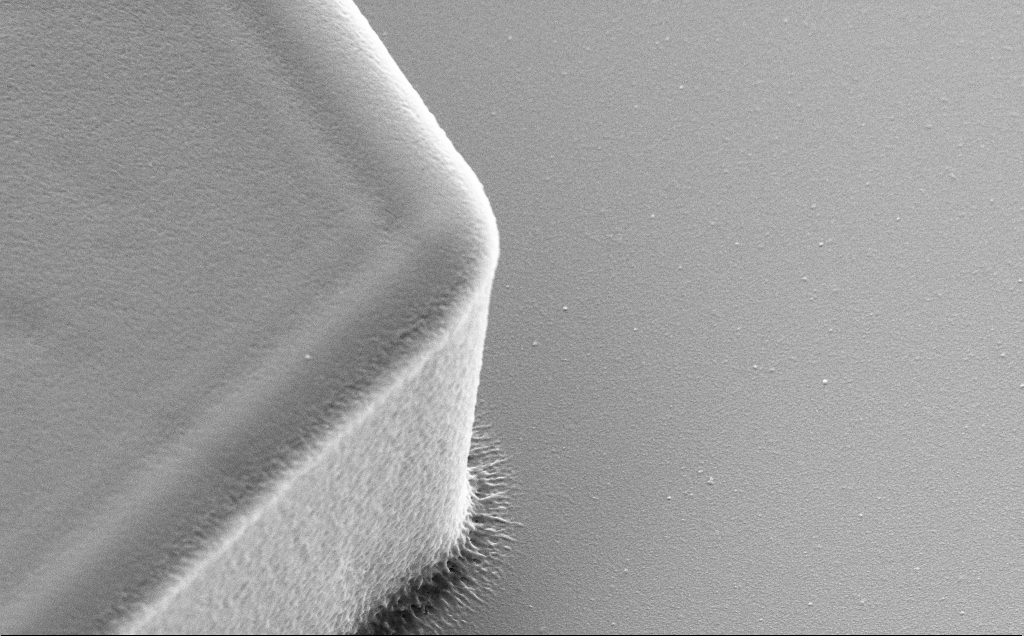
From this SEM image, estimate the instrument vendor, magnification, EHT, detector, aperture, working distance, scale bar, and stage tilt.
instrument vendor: Zeiss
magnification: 20.89 K X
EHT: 5 kV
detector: SE2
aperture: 30 µm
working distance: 11 mm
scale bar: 1000 nm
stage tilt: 30°